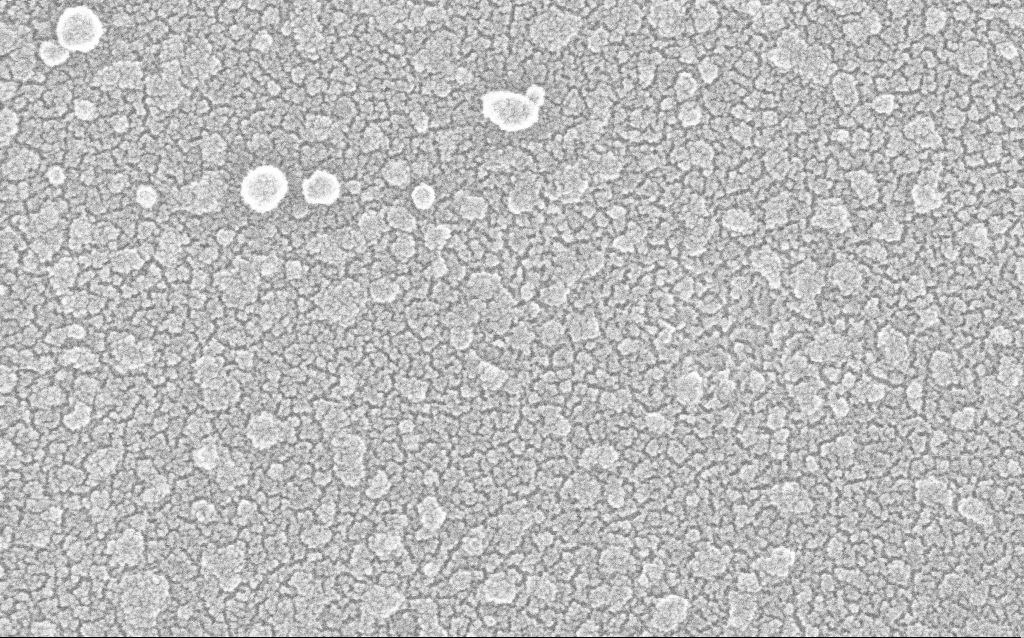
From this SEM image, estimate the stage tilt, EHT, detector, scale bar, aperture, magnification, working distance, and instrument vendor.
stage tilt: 0°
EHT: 20 kV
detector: InLens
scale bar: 100 nm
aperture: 30 µm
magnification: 300 K X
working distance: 1.8 mm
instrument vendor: Zeiss